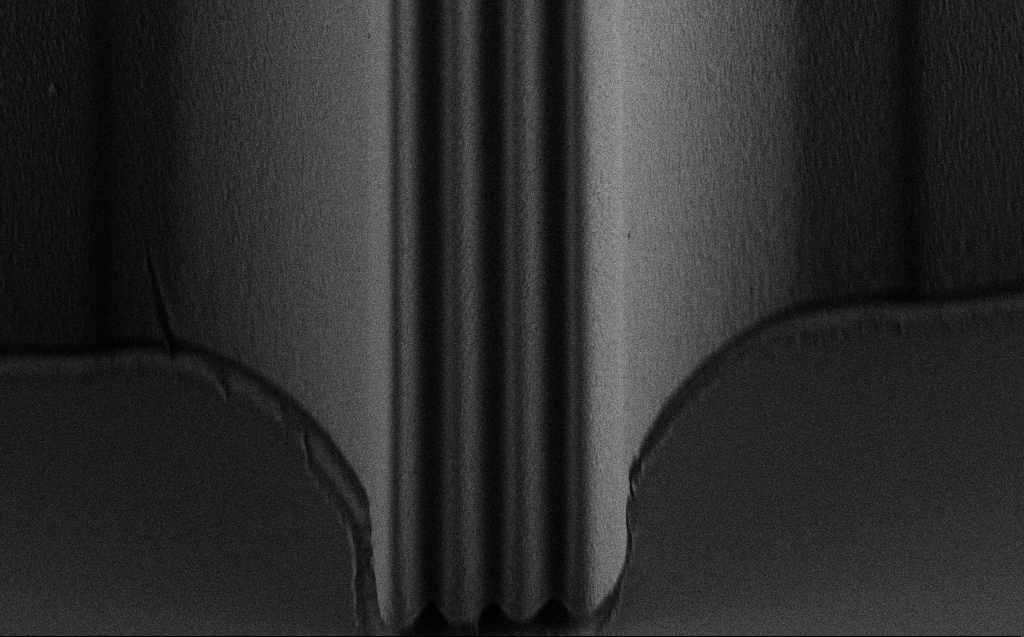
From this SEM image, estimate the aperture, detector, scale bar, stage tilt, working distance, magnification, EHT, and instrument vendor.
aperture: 30 µm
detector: SE2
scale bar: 10000 nm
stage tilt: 45°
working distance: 4 mm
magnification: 2.05 K X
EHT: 0.9 kV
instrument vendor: Zeiss